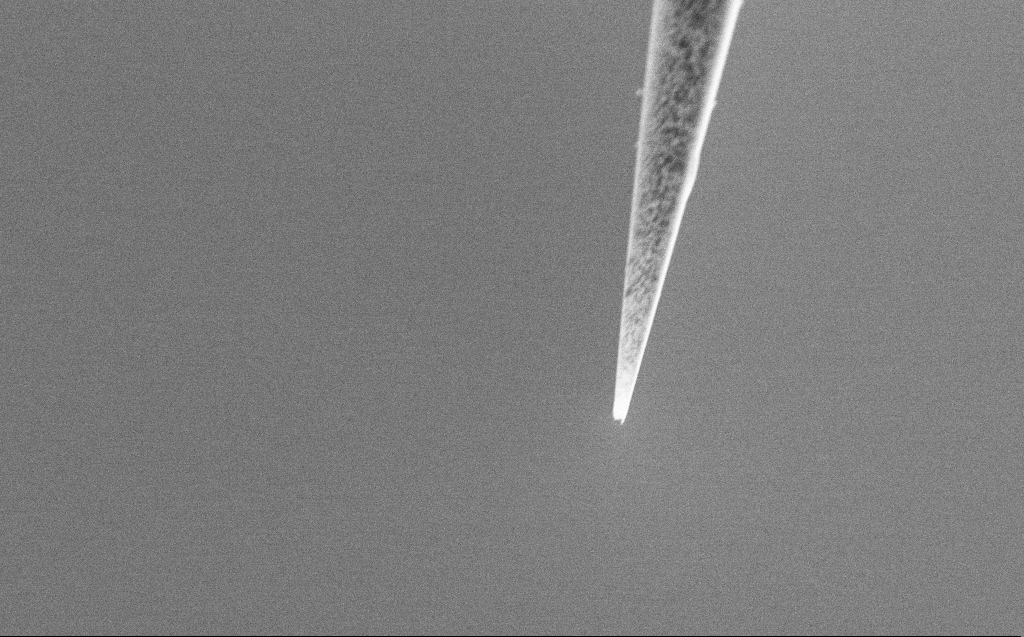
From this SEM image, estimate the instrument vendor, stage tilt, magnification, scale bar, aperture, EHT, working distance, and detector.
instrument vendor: Zeiss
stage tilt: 45°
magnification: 25 K X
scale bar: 2000 nm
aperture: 30 µm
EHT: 2 kV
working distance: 4 mm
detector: SE2